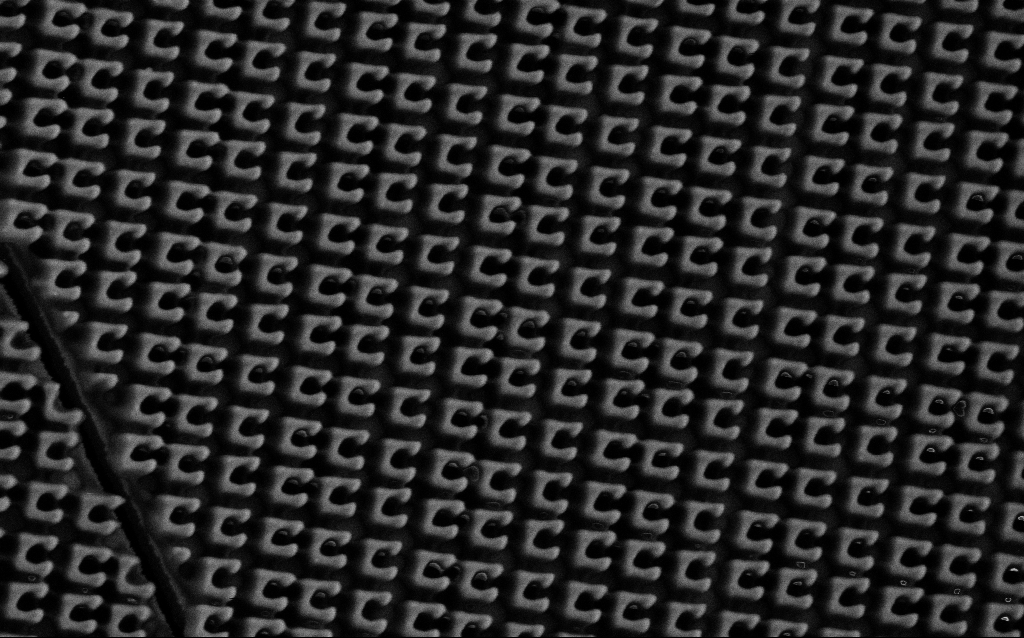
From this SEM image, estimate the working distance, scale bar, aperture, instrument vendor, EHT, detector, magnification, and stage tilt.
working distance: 6.2 mm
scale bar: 1000 nm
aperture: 30 µm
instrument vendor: Zeiss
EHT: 1.5 kV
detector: SE2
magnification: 41.32 K X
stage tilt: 0°